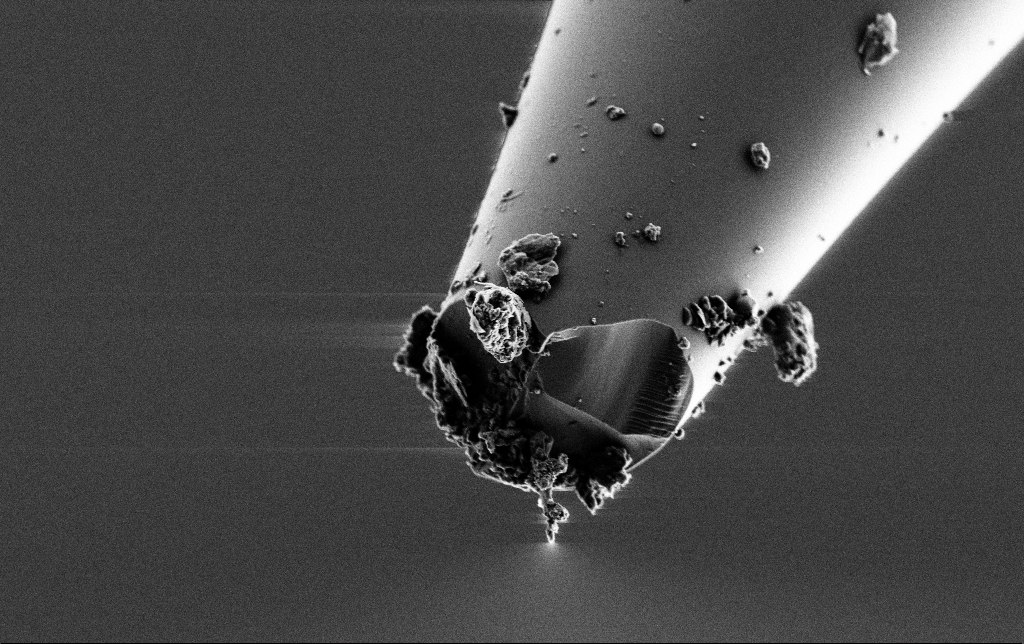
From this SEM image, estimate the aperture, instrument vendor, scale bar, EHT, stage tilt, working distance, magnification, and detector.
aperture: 30 µm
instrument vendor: Zeiss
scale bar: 2000 nm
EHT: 2 kV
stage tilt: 45°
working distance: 6 mm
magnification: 11.66 K X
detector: SE2